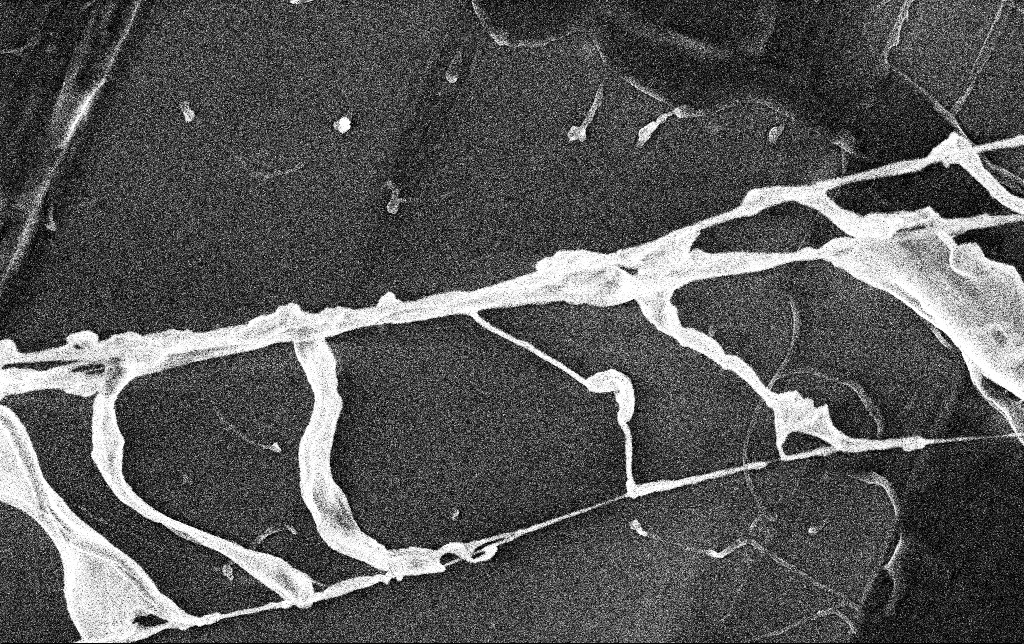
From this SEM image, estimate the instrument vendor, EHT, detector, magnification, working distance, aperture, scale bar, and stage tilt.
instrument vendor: Zeiss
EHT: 3 kV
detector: InLens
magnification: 23.28 K X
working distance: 3.5 mm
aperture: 30 µm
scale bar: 1000 nm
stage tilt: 0°